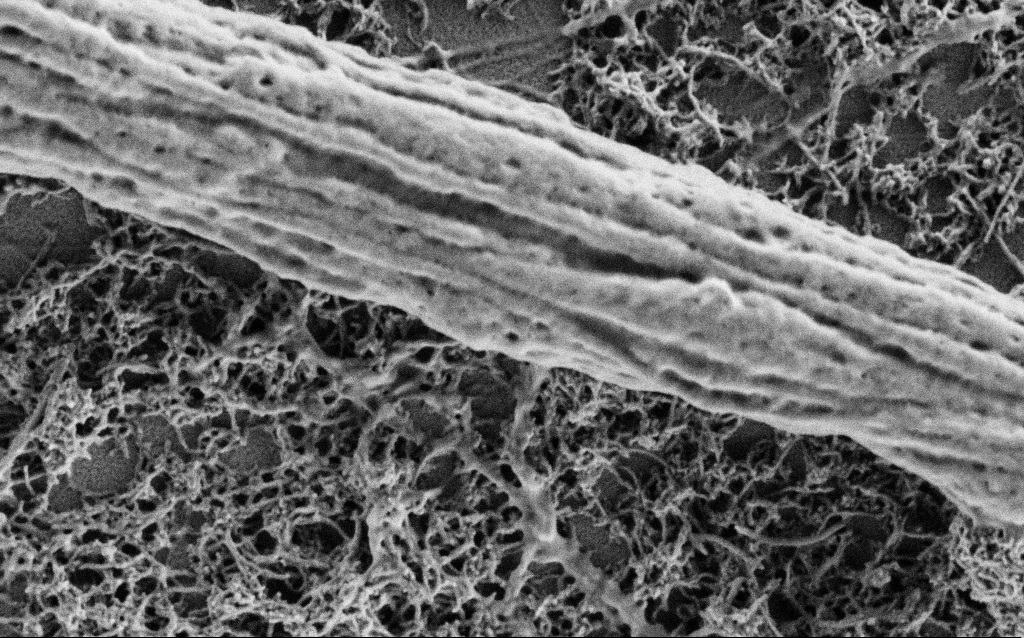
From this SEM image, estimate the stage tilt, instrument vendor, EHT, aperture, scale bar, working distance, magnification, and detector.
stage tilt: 0°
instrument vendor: Zeiss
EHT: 1 kV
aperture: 30 µm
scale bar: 1000 nm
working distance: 4 mm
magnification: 50 K X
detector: SE2